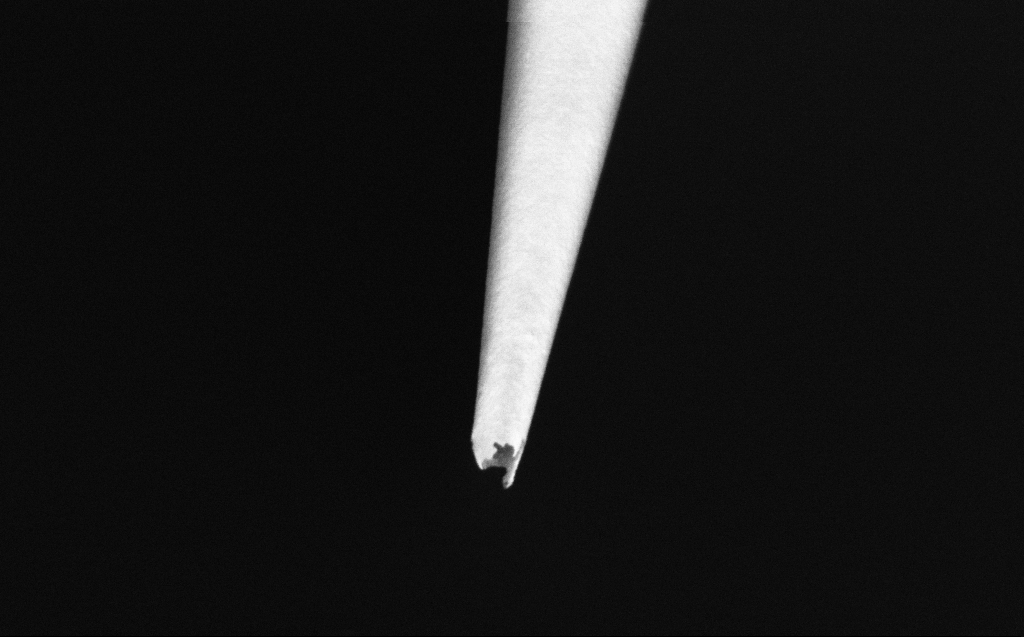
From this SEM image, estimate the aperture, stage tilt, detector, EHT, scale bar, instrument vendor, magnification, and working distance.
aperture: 30 µm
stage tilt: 45°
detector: InLens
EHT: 2 kV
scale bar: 200 nm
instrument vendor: Zeiss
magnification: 100 K X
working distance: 4 mm